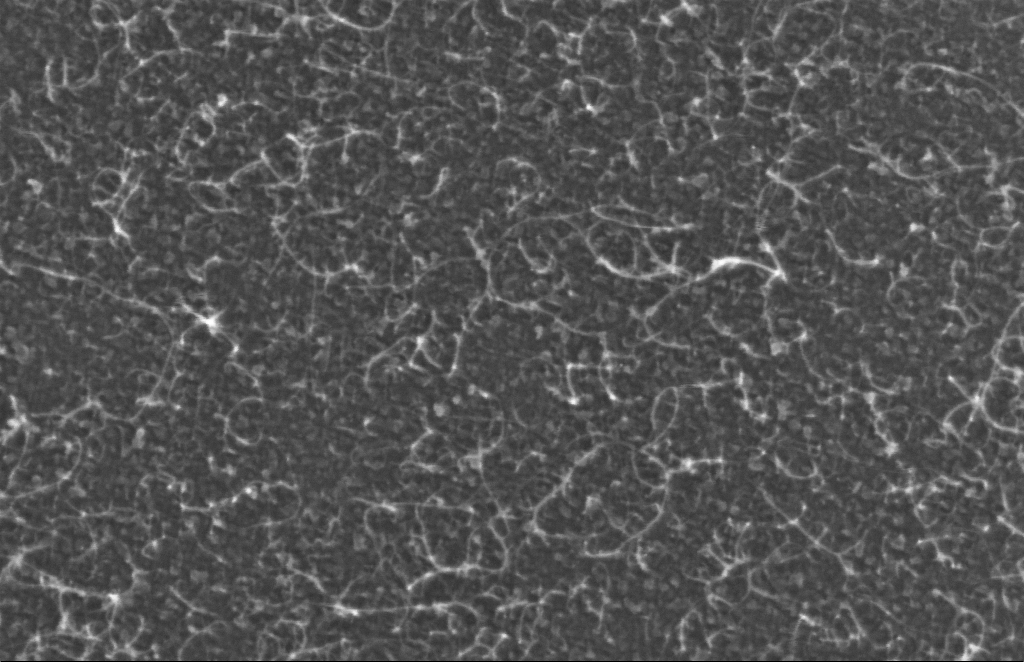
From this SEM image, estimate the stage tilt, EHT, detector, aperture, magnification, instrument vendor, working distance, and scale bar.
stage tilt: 0°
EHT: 5 kV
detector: InLens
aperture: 30 µm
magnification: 343.02 K X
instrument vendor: Zeiss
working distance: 4 mm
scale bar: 100 nm